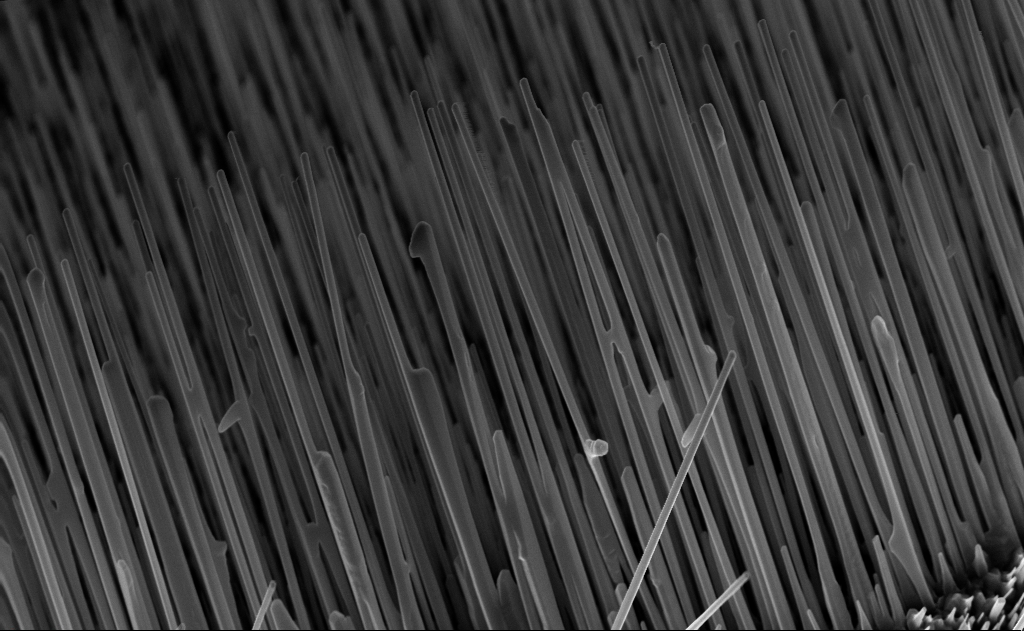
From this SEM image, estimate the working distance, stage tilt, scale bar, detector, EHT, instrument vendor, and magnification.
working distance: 5 mm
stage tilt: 0°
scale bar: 2000 nm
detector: InLens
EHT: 10 kV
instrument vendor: Zeiss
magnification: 20 K X